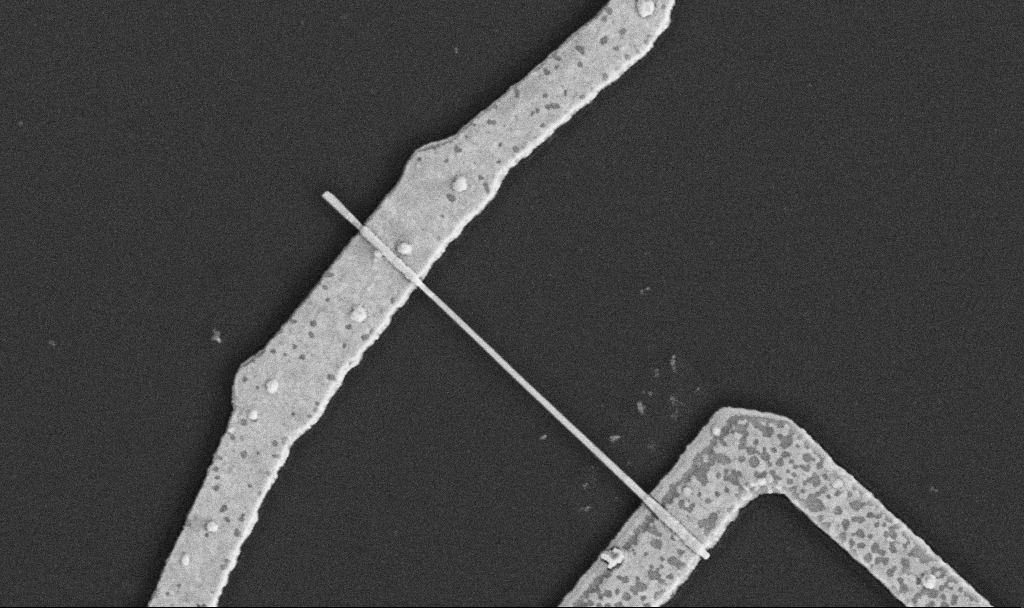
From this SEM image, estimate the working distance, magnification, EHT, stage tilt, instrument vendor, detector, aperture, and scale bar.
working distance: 10.6 mm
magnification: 30 K X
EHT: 5 kV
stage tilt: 0°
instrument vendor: Zeiss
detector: SE2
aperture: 30 µm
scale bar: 1000 nm